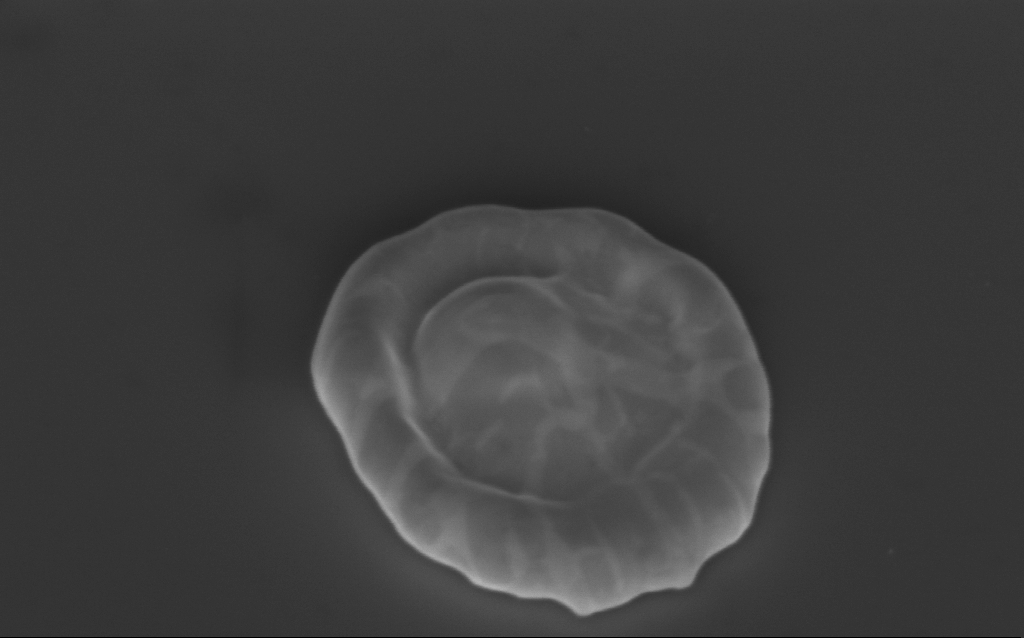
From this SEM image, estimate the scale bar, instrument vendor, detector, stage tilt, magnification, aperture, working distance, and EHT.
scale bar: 200 nm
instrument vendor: Zeiss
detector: InLens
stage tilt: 40°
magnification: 84.27 K X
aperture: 30 µm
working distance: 5 mm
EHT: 3 kV